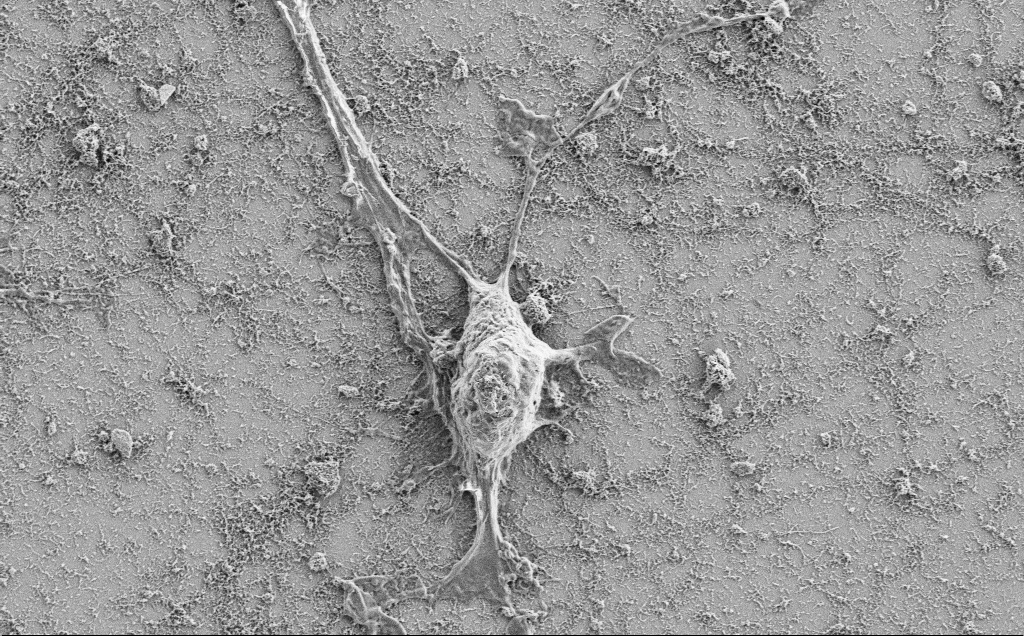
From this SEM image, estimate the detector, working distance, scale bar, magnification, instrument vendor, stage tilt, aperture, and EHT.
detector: SE2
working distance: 7.1 mm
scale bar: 10000 nm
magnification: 5 K X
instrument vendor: Zeiss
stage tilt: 0°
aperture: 30 µm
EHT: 2 kV